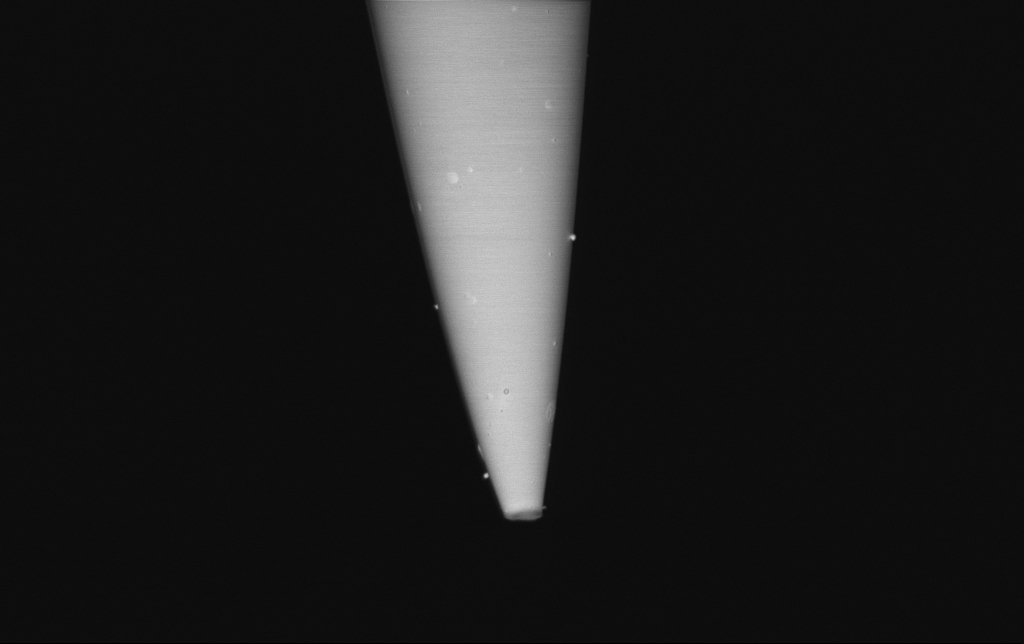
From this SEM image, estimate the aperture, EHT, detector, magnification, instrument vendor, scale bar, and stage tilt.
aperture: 30 µm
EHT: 1 kV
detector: InLens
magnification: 10 K X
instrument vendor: Zeiss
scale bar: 2000 nm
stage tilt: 0°